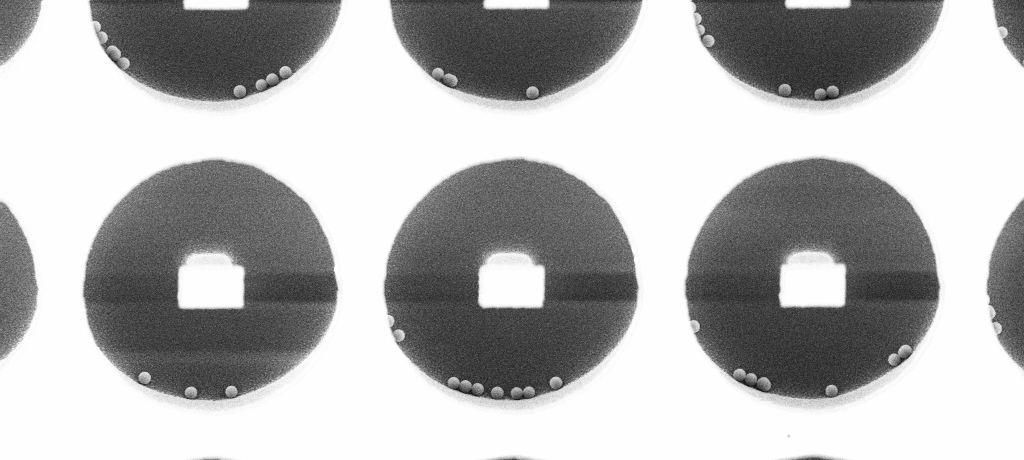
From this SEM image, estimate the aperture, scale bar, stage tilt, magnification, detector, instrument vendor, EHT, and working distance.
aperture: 30 µm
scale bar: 2000 nm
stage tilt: -0°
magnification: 7.92 K X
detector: InLens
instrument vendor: Zeiss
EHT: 5 kV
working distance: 3.3 mm